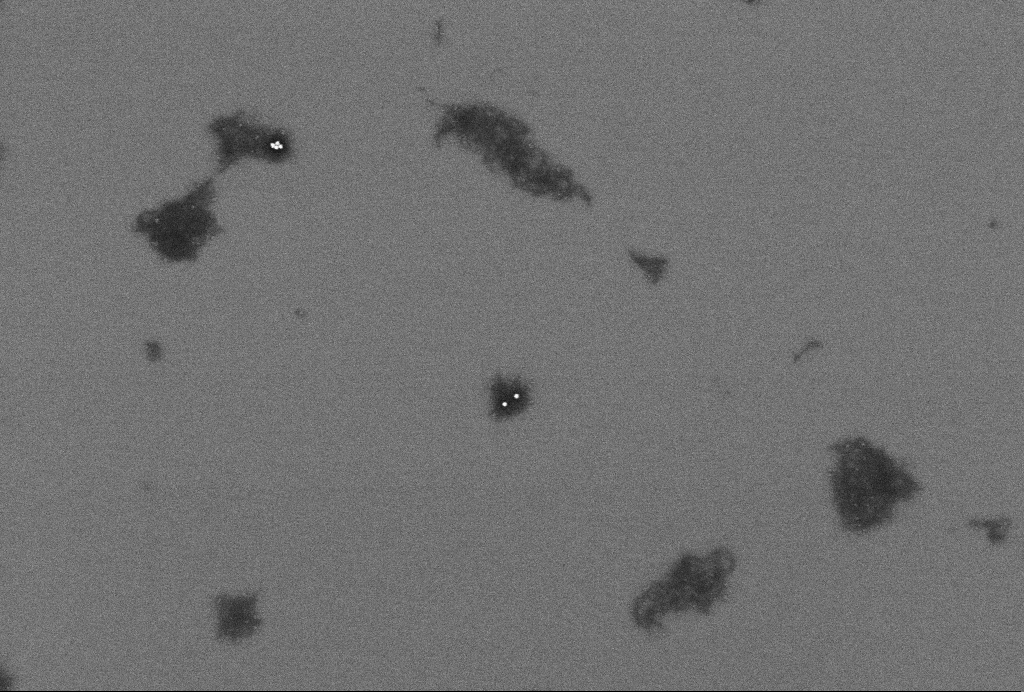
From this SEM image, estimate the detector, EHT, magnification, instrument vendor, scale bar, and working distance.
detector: InLens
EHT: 2 kV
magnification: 62.66 K X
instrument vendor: Zeiss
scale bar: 200 nm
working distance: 3.3 mm